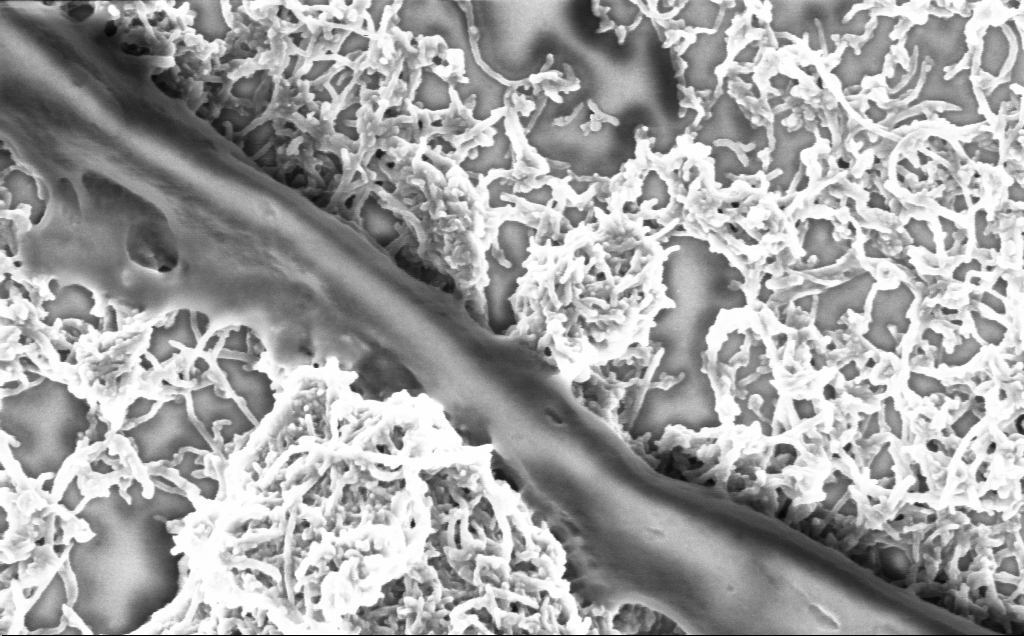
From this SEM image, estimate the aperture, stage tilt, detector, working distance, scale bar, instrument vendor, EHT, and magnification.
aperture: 30 µm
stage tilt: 0°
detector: InLens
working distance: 7.1 mm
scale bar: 200 nm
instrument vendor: Zeiss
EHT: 2 kV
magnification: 75 K X